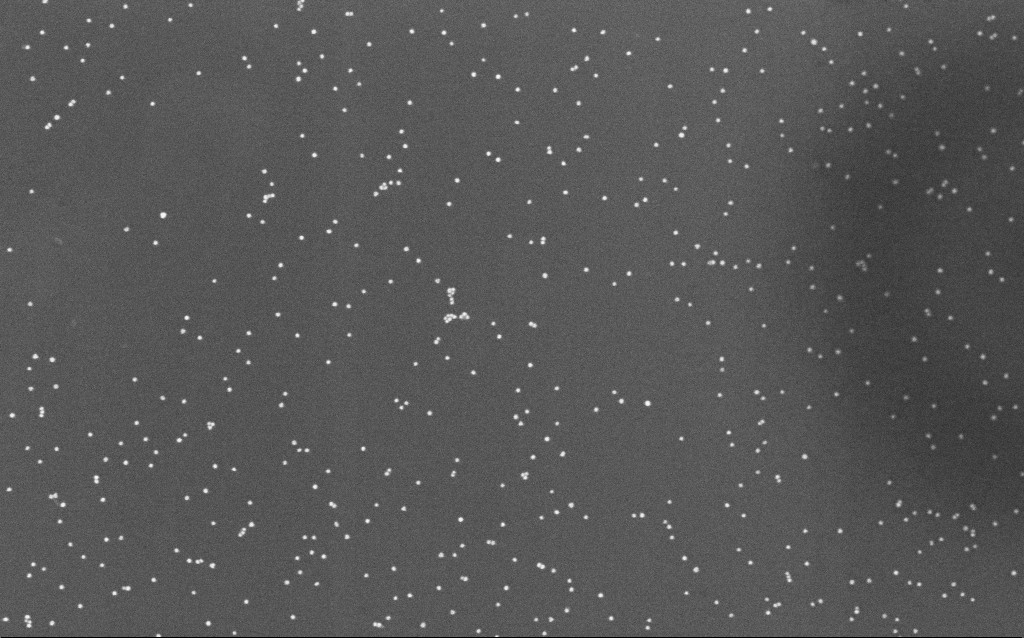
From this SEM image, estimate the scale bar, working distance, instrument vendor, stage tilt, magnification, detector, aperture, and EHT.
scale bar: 200 nm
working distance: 6.6 mm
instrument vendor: Zeiss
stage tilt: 0°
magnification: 100 K X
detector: InLens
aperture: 30 µm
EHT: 10 kV